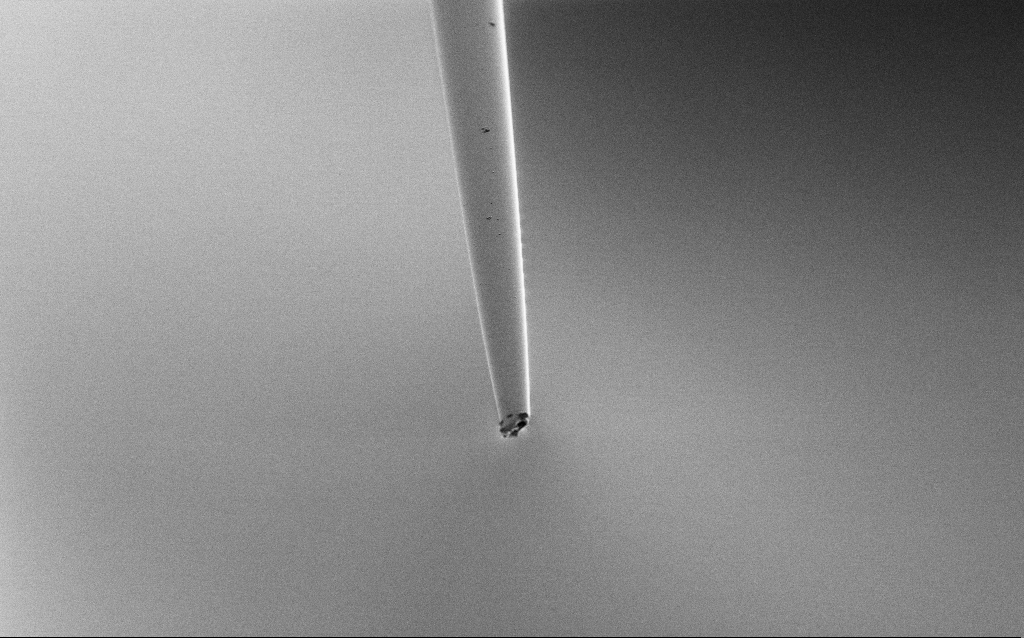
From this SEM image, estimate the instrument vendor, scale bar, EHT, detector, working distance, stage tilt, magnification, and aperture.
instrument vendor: Zeiss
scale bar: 20000 nm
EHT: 2 kV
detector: SE2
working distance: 6 mm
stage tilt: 45°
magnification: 1 K X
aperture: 30 µm